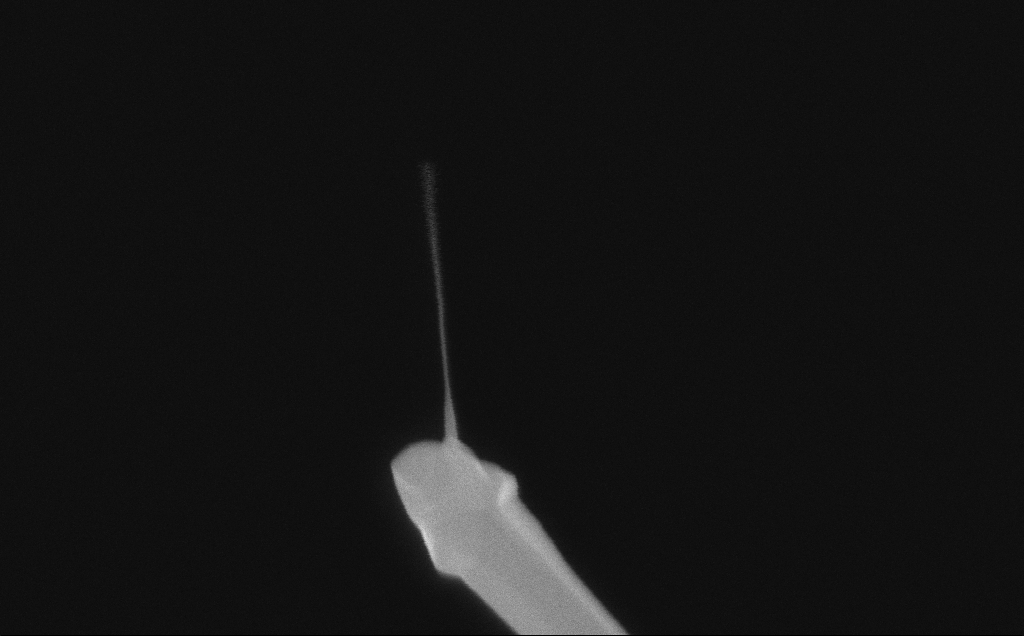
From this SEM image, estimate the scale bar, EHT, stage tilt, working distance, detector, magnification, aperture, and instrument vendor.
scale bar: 200 nm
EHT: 10 kV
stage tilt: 0°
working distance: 6 mm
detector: InLens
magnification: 189.64 K X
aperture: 30 µm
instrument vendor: Zeiss